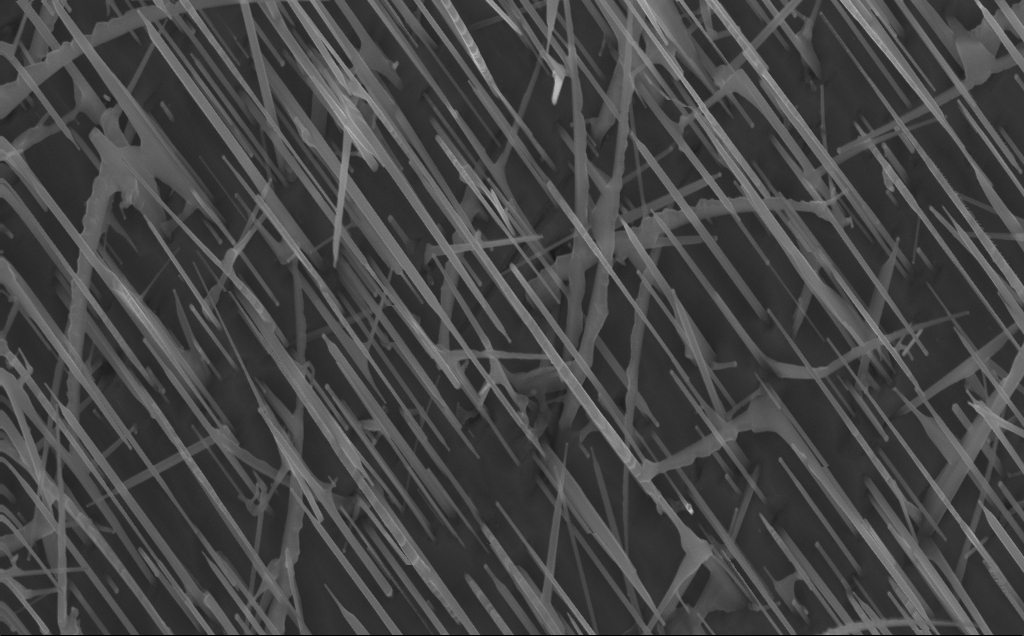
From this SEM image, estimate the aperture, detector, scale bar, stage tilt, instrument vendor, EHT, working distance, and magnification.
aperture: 30 µm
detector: InLens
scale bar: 1000 nm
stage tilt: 0°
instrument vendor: Zeiss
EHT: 10 kV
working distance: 4 mm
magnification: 40 K X